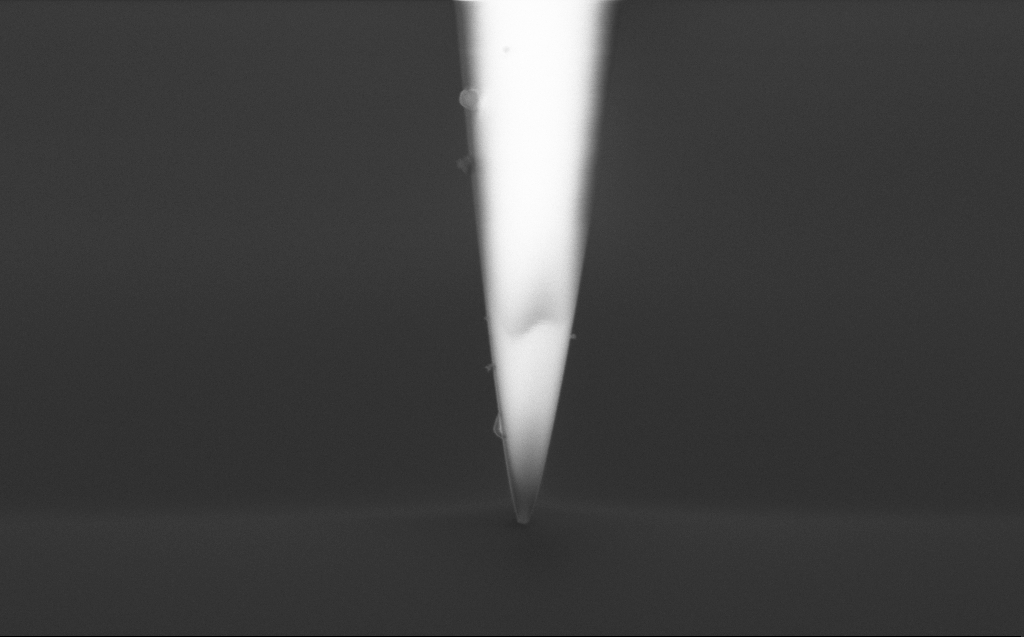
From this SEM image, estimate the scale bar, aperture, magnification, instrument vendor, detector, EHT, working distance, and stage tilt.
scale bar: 1000 nm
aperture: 30 µm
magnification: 44.35 K X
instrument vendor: Zeiss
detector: InLens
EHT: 3 kV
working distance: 3 mm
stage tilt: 45.1°